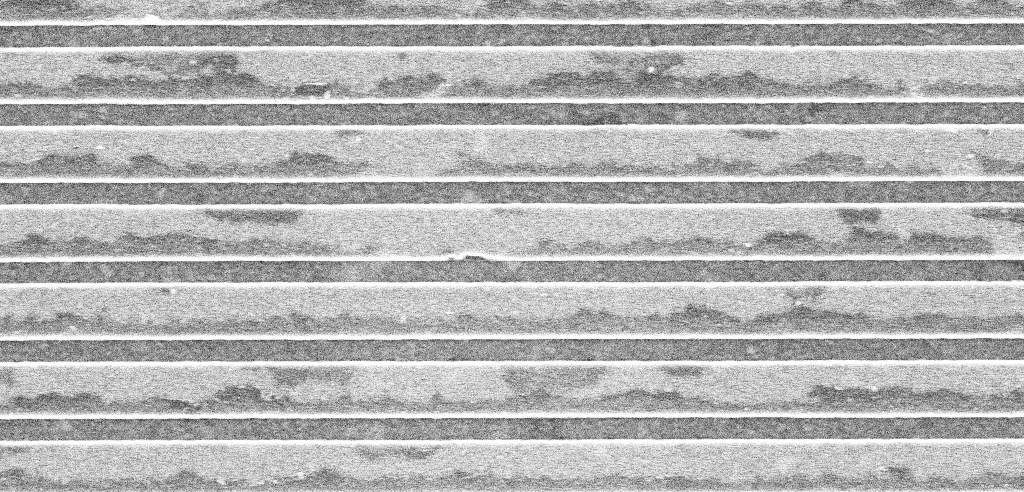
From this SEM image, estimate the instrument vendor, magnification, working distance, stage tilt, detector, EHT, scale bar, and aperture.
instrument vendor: Zeiss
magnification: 41.62 K X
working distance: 3.1 mm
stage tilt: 0°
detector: InLens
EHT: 5 kV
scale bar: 1000 nm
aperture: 30 µm